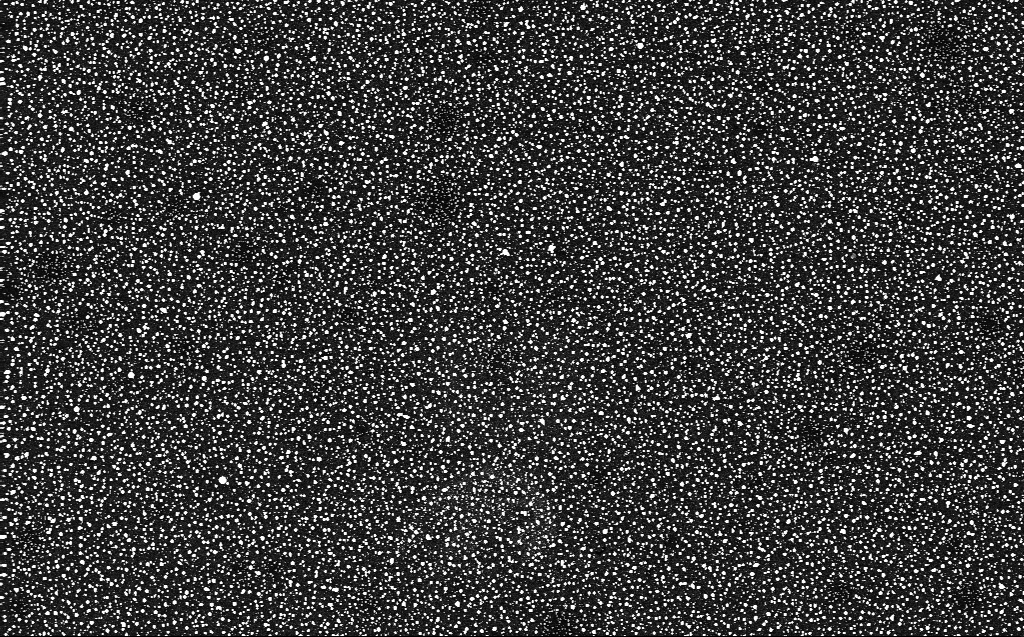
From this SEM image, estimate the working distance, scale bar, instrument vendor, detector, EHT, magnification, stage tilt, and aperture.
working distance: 3 mm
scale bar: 10000 nm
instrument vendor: Zeiss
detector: InLens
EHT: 10 kV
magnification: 6.32 K X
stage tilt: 0°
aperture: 30 µm